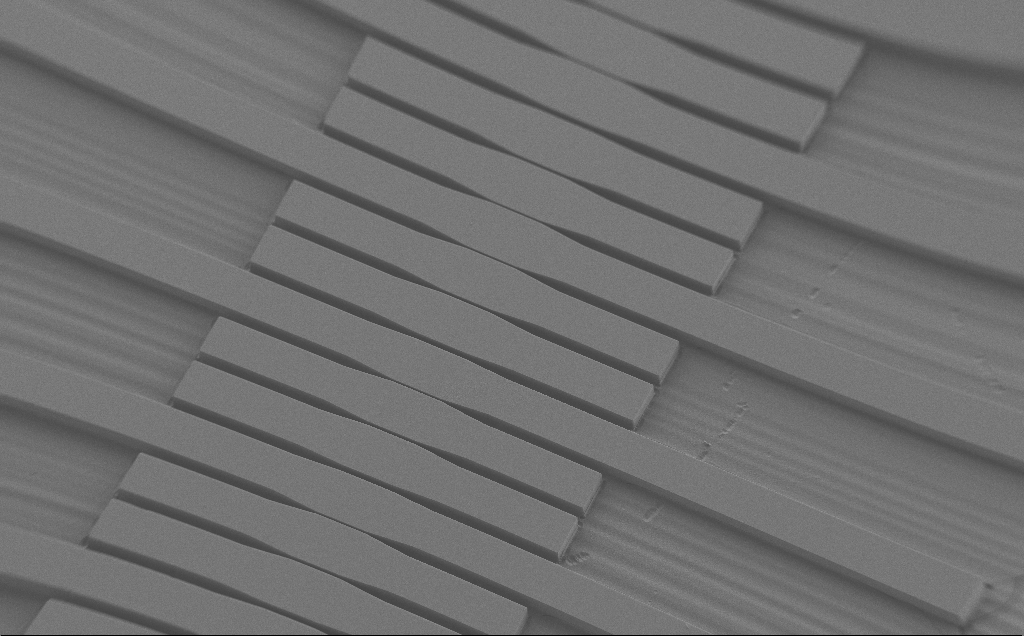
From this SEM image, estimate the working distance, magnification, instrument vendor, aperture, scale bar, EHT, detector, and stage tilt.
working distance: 6 mm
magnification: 0.194 K X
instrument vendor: Zeiss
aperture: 30 µm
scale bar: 100000 nm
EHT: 1.2 kV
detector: SE2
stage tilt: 30°